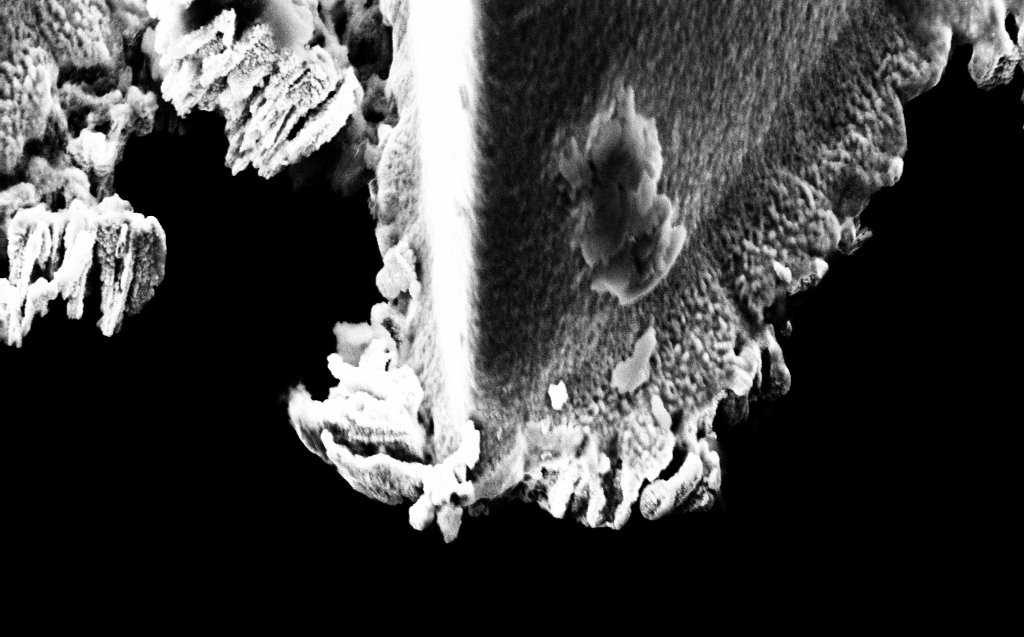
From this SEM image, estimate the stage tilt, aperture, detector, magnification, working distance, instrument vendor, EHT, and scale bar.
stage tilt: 45°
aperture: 30 µm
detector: InLens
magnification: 29.57 K X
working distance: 6 mm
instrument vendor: Zeiss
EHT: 5 kV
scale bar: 2000 nm